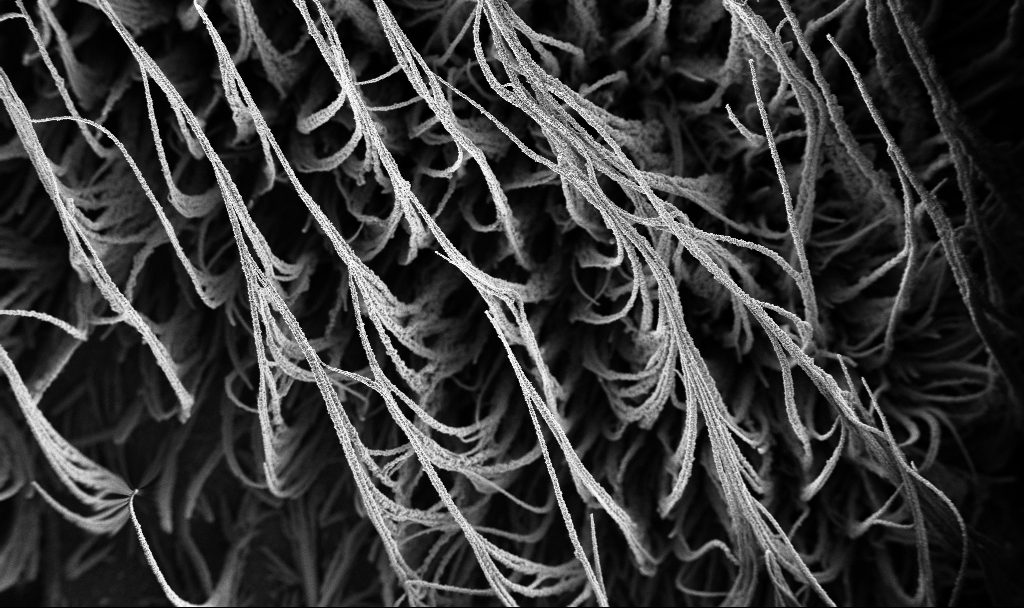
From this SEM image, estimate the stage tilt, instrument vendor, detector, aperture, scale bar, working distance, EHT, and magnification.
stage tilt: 0°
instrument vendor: Zeiss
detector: InLens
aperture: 30 µm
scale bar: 100000 nm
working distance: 2.9 mm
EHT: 3 kV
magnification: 0.15 K X